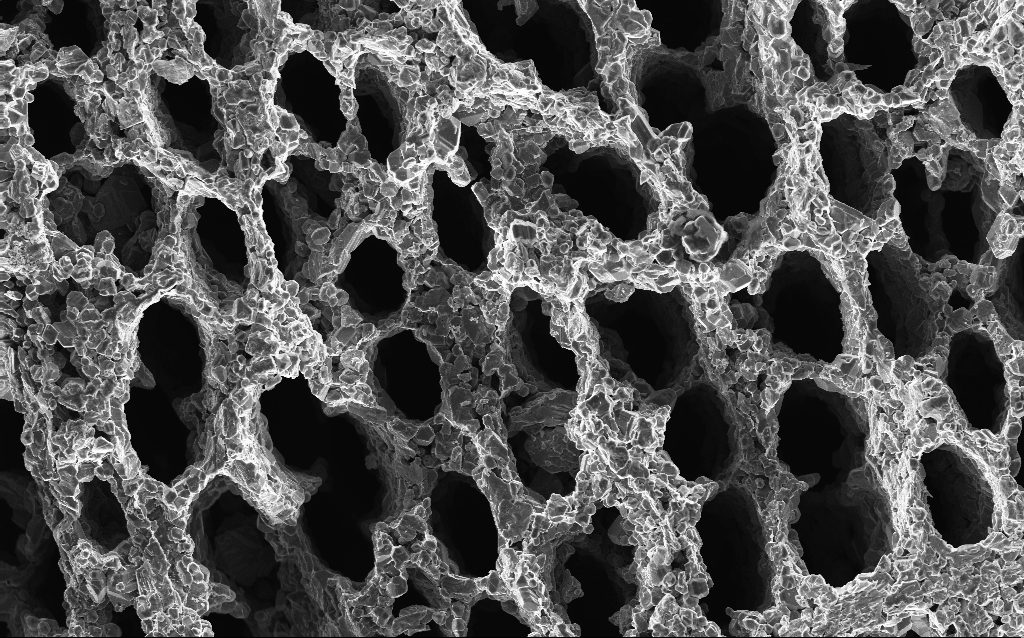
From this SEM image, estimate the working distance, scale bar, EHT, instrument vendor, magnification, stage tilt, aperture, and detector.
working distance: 3 mm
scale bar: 20000 nm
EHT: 10 kV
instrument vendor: Zeiss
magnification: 1 K X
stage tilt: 0°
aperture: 30 µm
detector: InLens